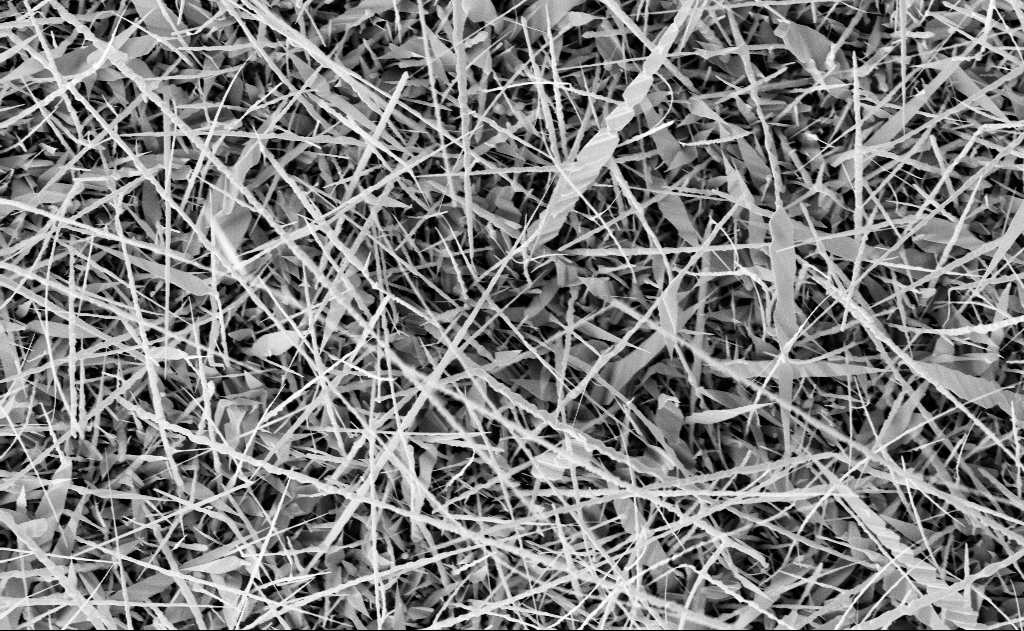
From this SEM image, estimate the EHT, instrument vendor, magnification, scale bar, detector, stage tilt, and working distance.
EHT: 10 kV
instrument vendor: Zeiss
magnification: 10 K X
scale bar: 2000 nm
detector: InLens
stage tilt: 0°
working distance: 19 mm